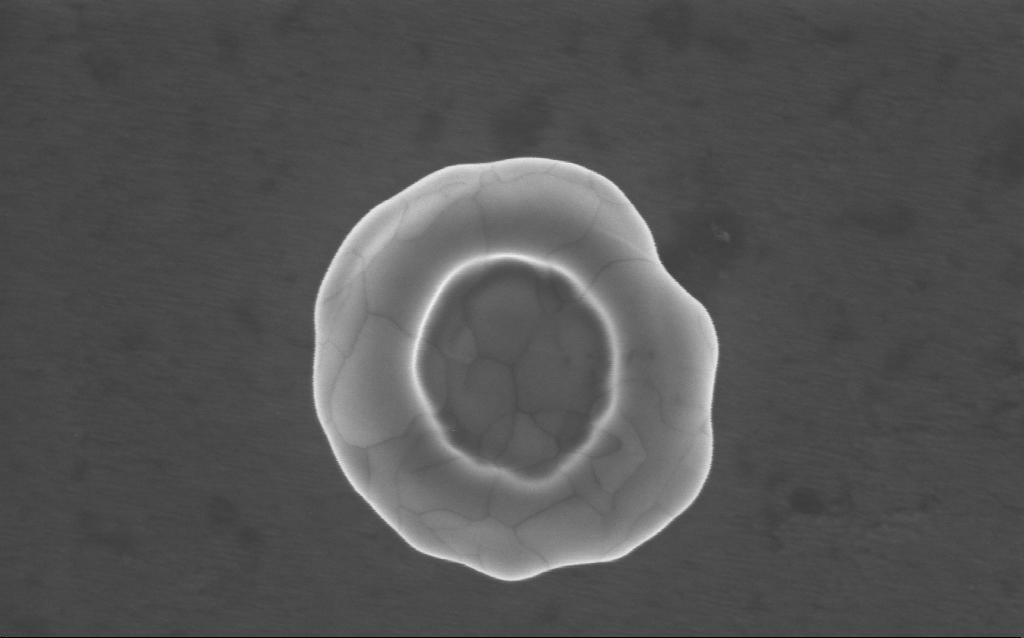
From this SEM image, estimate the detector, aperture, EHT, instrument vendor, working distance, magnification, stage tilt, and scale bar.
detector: InLens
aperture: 30 µm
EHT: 5 kV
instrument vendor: Zeiss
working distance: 4 mm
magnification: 152 K X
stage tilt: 0°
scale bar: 200 nm